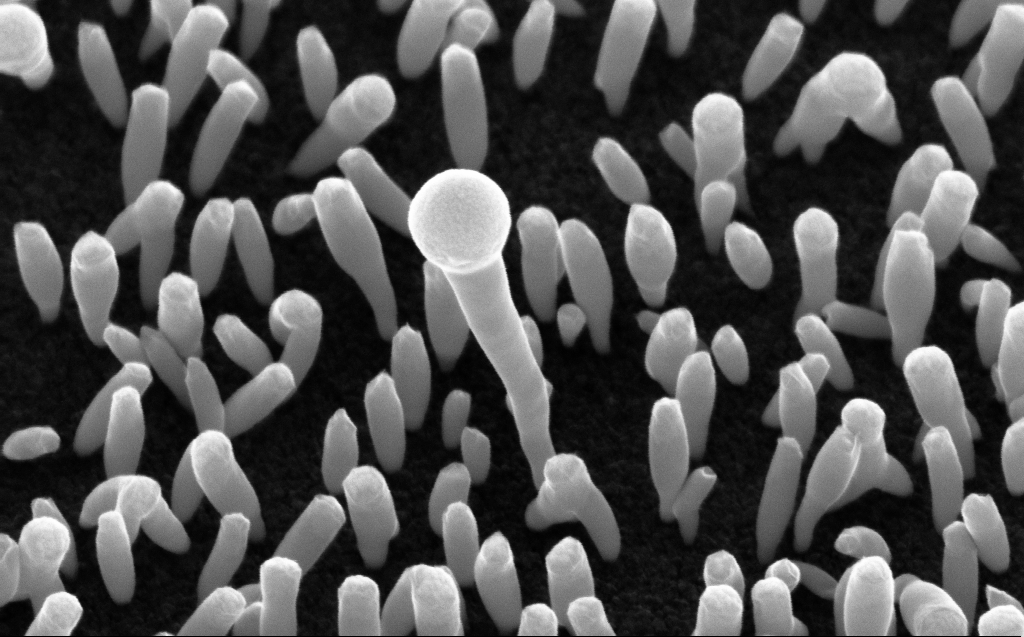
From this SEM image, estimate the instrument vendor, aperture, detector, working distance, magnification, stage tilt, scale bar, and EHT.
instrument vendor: Zeiss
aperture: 30 µm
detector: InLens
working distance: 5 mm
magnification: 121.84 K X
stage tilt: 45°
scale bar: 200 nm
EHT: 10 kV